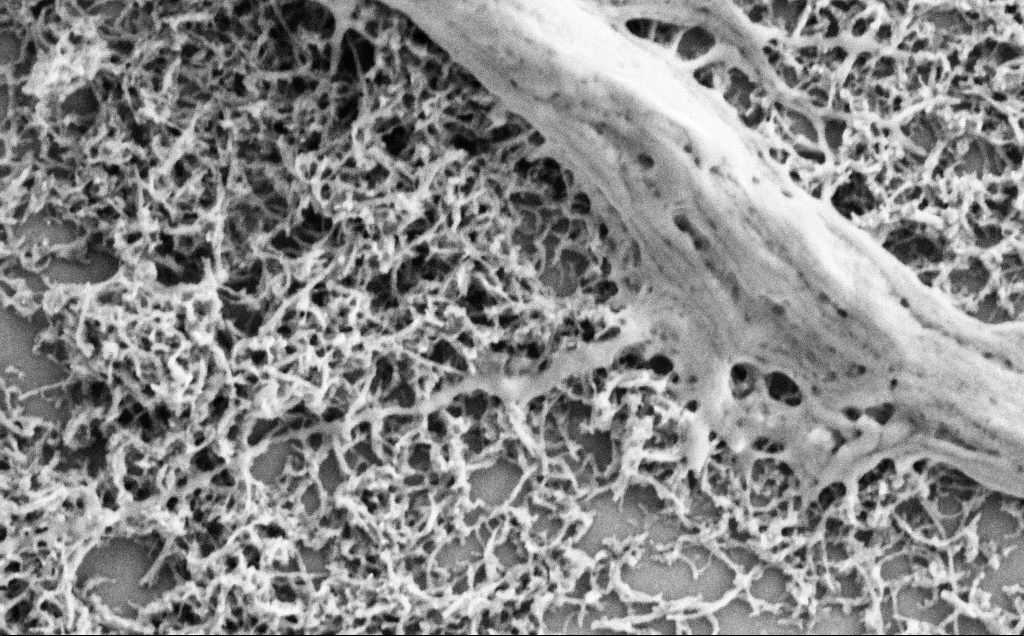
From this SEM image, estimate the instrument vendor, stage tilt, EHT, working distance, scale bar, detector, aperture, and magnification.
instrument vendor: Zeiss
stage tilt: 0°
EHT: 2 kV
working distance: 7.1 mm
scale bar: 1000 nm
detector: SE2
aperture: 30 µm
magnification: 50 K X